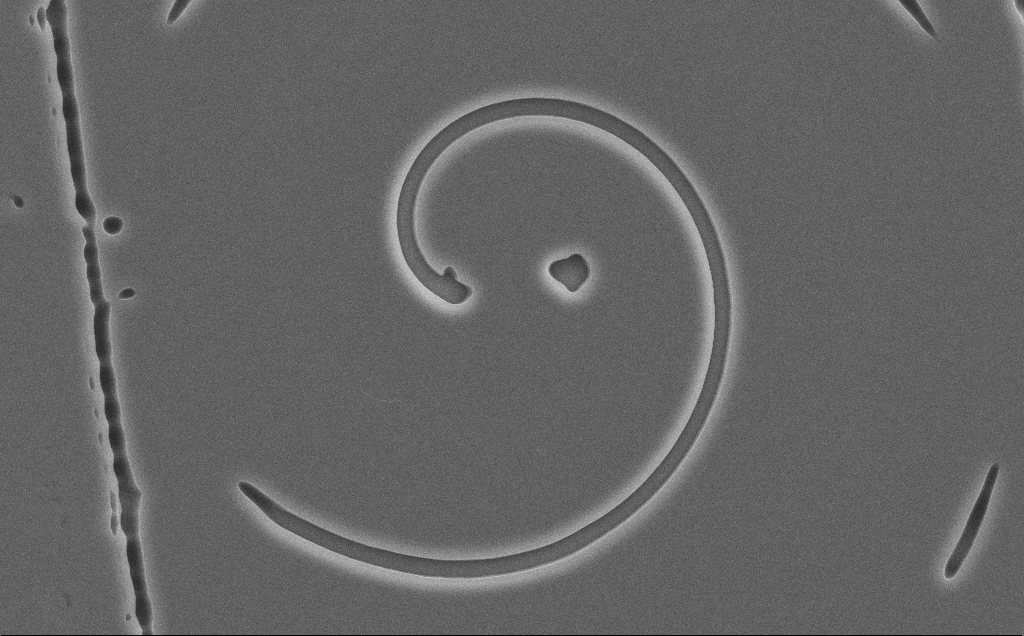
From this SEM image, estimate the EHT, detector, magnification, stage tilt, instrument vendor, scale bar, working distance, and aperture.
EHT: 10 kV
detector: SE2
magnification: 4.17 K X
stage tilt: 0°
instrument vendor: Zeiss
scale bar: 10000 nm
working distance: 12 mm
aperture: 30 µm